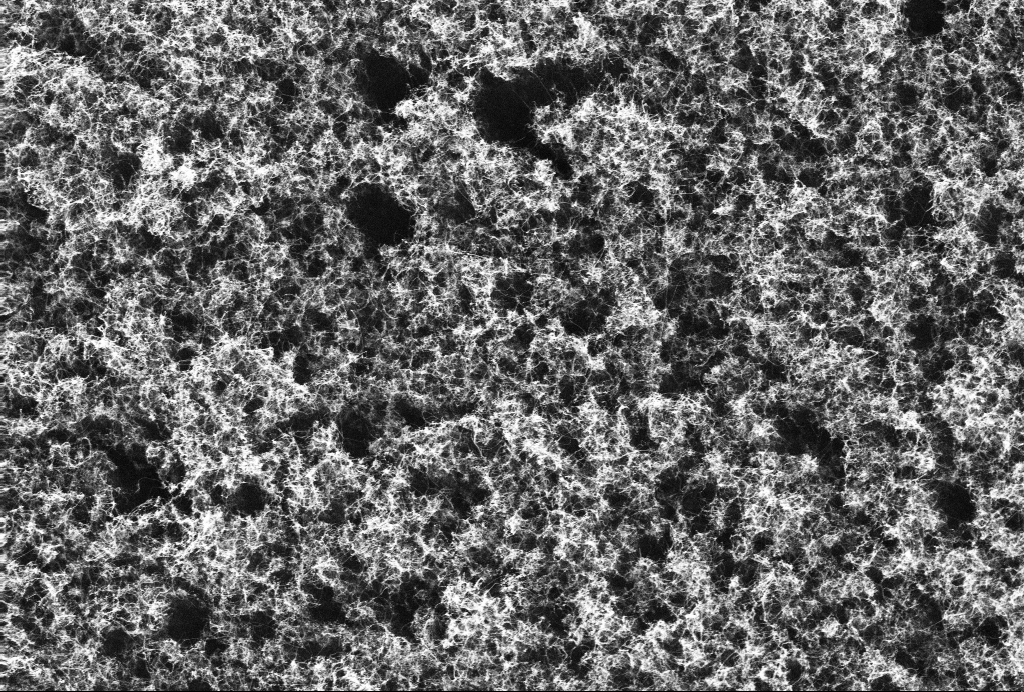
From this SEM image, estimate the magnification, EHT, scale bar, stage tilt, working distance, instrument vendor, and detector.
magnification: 43.38 K X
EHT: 10 kV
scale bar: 1000 nm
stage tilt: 0°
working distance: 2.9 mm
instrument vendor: Zeiss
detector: InLens